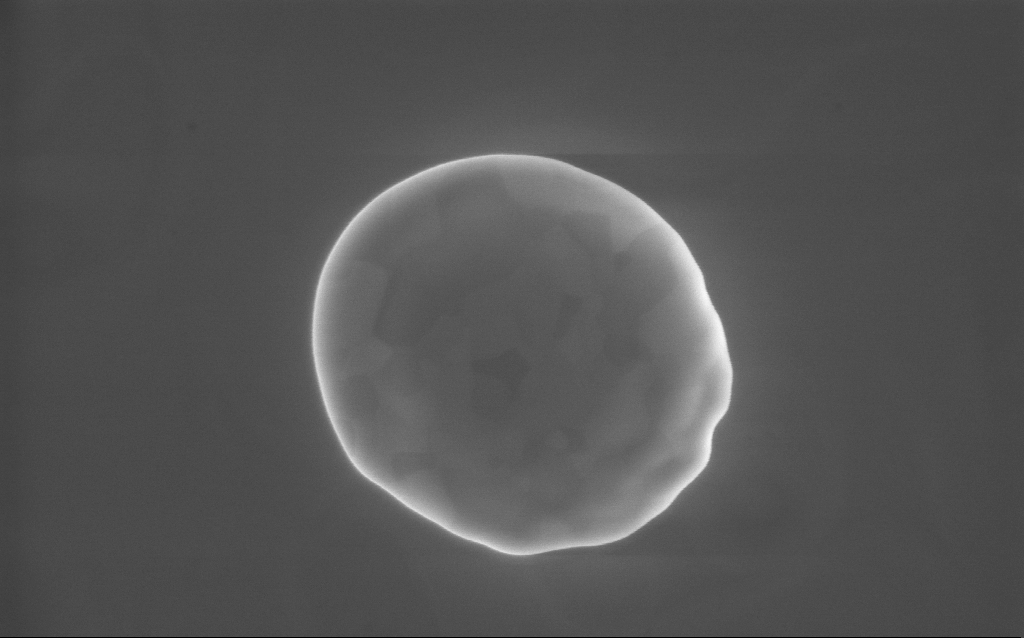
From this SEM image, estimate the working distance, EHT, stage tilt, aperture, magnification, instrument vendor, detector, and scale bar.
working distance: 2 mm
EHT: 10 kV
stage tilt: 0°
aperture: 30 µm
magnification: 63.86 K X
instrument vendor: Zeiss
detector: InLens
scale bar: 1000 nm